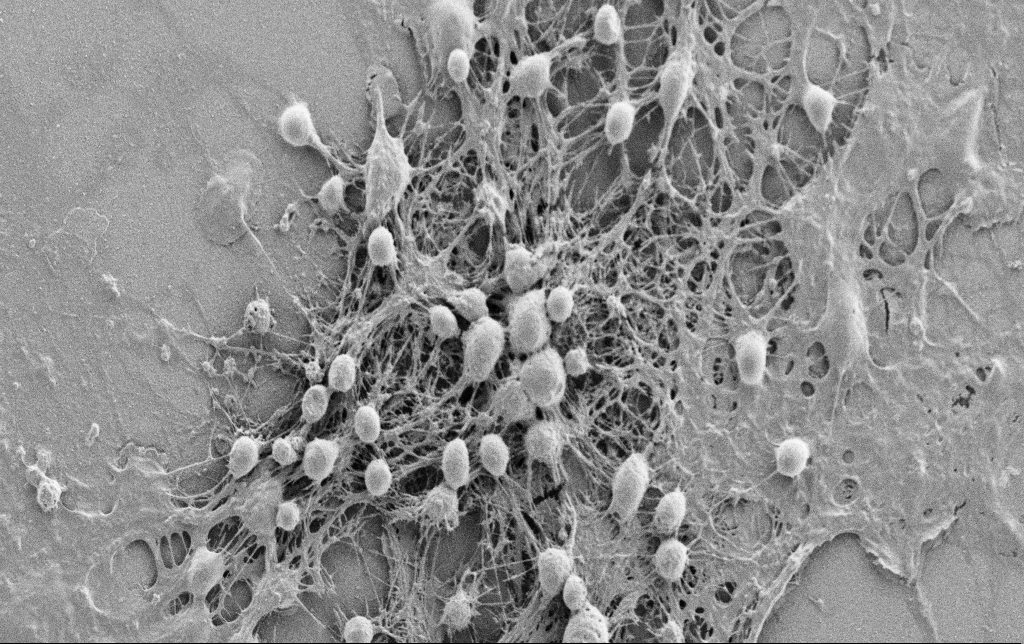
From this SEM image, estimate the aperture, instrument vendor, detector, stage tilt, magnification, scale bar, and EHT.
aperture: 30 µm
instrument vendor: Zeiss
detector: SE2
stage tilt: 0°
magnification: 2.5 K X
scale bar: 20000 nm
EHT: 0.9 kV